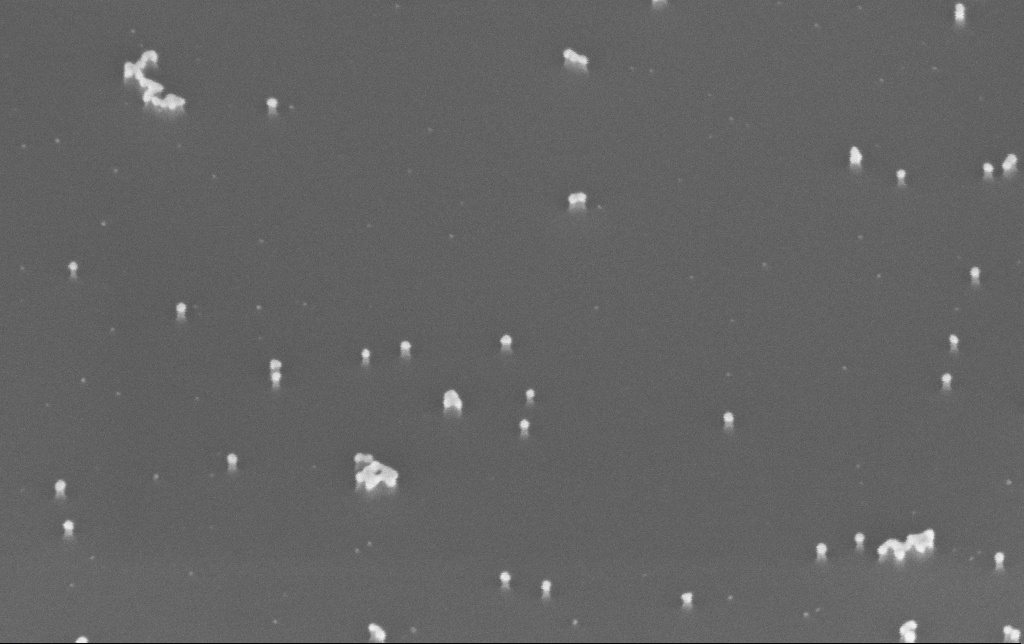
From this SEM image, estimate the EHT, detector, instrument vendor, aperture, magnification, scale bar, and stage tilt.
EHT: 10 kV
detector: InLens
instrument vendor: Zeiss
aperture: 30 µm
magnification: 200 K X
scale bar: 100 nm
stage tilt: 45°